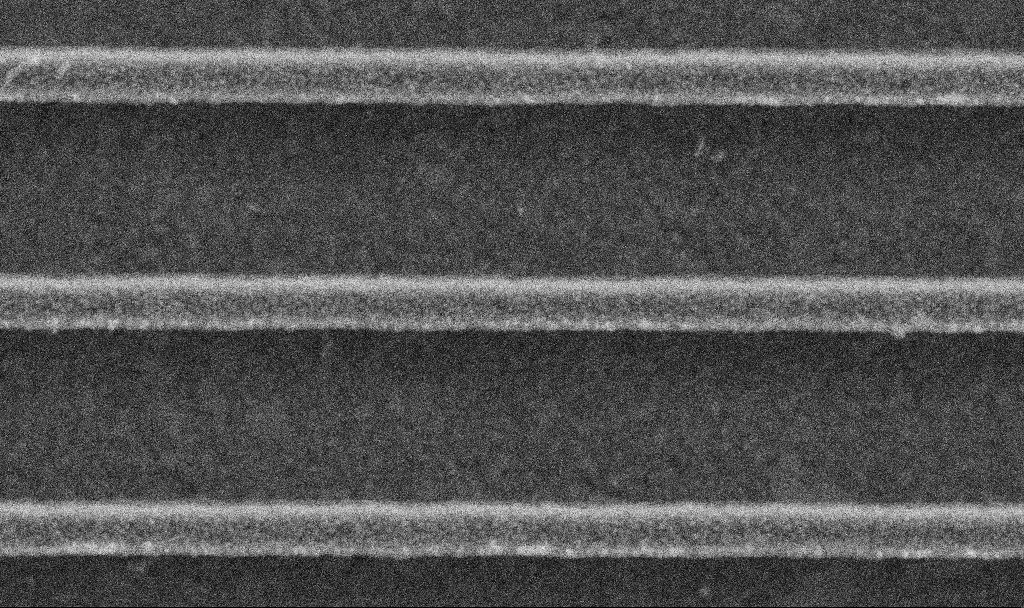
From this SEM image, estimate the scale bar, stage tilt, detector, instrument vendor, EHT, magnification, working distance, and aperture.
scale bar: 200 nm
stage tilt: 0°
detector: SE2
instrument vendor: Zeiss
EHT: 5 kV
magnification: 139.02 K X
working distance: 3 mm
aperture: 30 µm